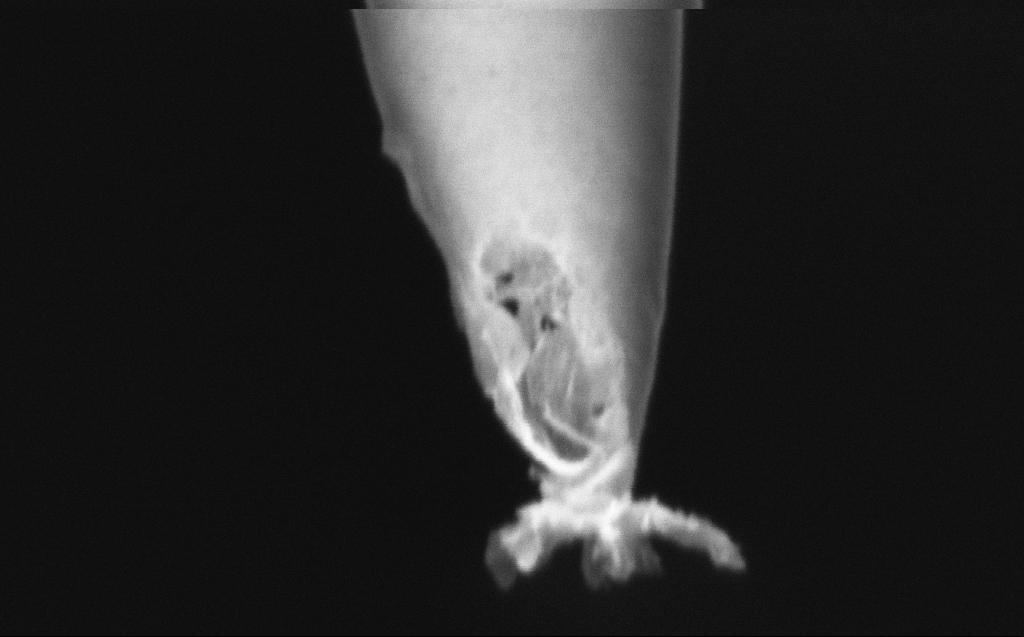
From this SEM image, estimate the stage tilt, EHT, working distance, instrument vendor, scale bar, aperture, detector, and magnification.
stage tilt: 45°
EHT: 2 kV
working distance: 6 mm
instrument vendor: Zeiss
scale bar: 200 nm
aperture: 30 µm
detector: InLens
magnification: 250 K X